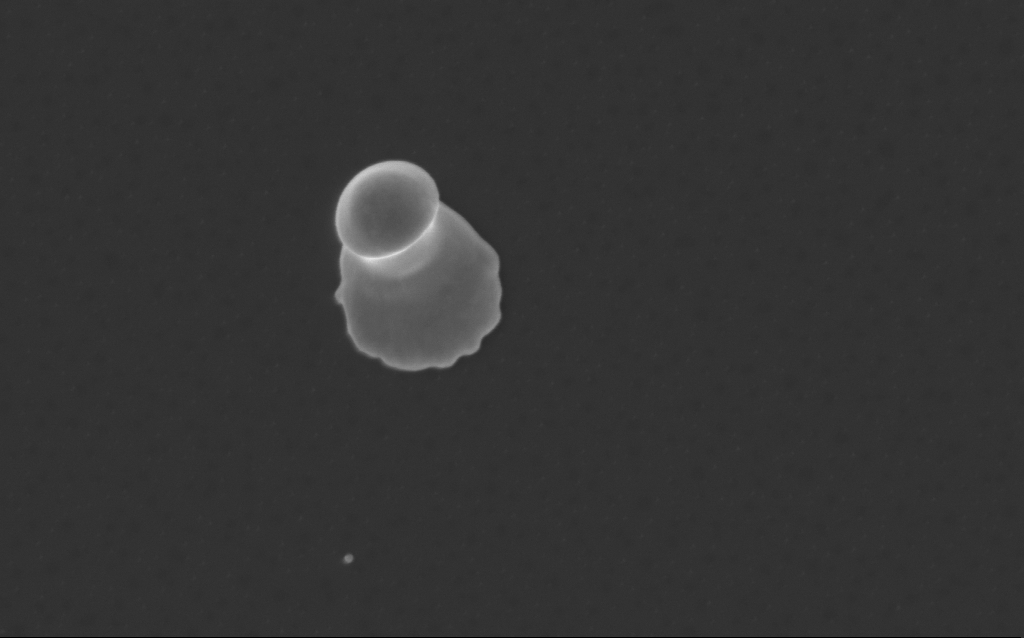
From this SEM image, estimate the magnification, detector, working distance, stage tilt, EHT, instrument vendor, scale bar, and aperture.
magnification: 55.08 K X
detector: InLens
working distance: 4 mm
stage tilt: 0°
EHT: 3 kV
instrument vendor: Zeiss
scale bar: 1000 nm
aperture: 30 µm